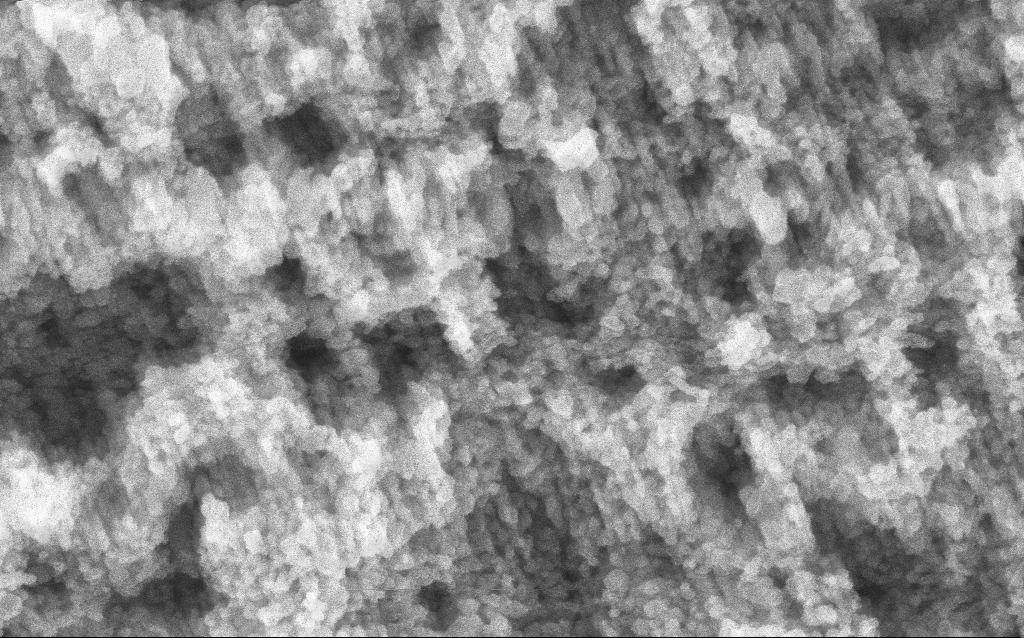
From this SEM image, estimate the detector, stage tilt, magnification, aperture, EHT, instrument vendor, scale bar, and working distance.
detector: InLens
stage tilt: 0°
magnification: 162.39 K X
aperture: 30 µm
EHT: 5 kV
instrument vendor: Zeiss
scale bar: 100 nm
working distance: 4.4 mm